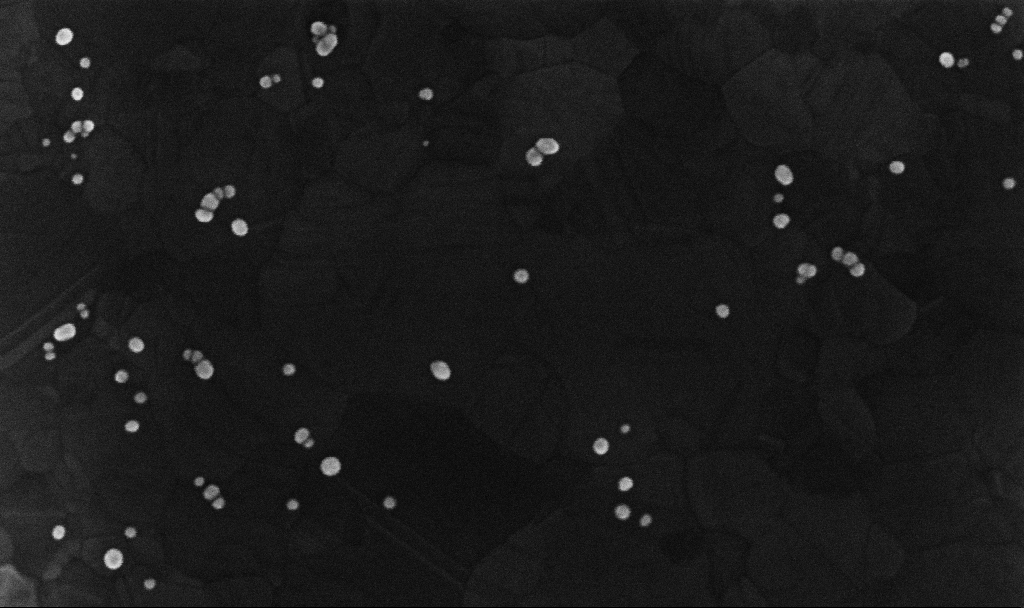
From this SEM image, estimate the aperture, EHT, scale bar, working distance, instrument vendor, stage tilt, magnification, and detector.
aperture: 30 µm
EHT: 10 kV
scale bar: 200 nm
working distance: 3.4 mm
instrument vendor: Zeiss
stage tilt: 0°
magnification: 229.63 K X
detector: InLens